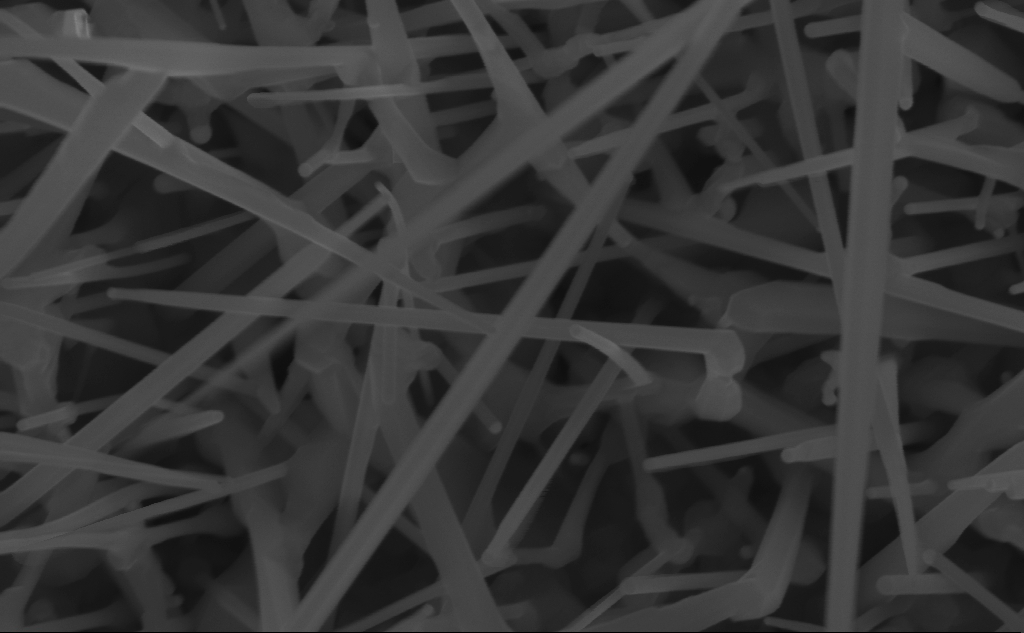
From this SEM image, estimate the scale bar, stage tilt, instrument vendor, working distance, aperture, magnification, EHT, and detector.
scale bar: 200 nm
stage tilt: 0°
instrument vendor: Zeiss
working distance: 7 mm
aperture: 30 µm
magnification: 132.69 K X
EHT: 10 kV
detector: InLens